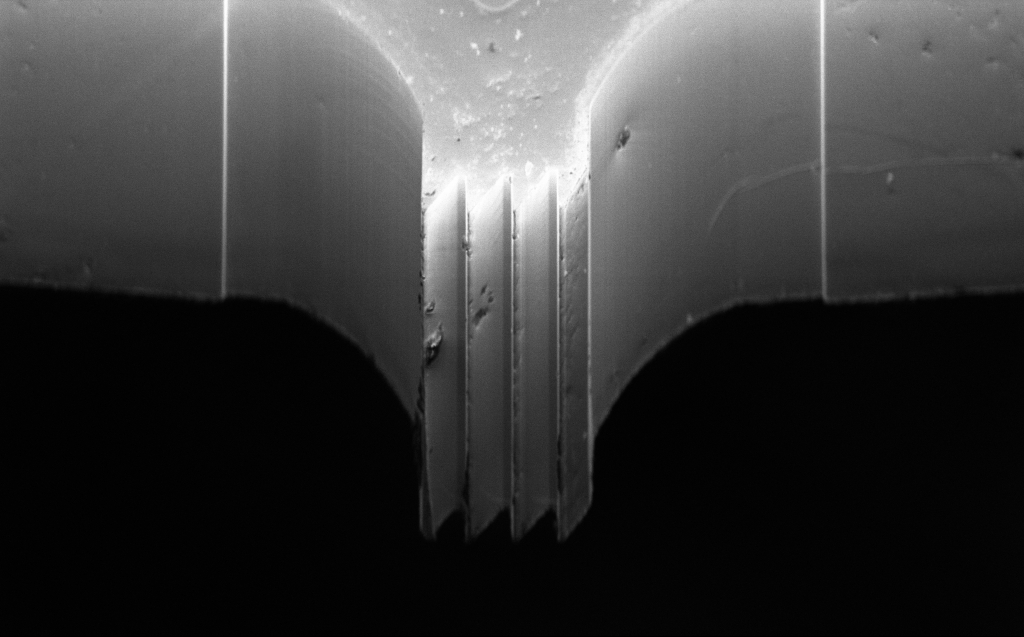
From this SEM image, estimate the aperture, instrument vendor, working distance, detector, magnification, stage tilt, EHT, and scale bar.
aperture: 30 µm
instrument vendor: Zeiss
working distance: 8 mm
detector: InLens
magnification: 1.48 K X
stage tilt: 45°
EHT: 3 kV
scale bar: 10000 nm